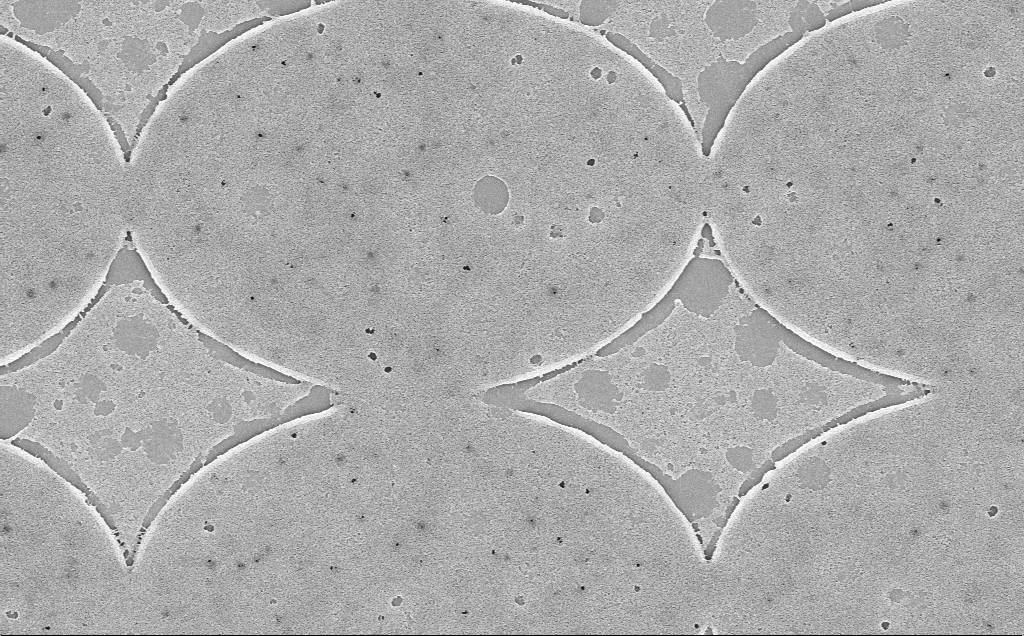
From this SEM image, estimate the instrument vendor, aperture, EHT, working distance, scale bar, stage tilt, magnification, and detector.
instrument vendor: Zeiss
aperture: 30 µm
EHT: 5 kV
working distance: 6 mm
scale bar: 2000 nm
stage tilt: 0°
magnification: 21.32 K X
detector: InLens